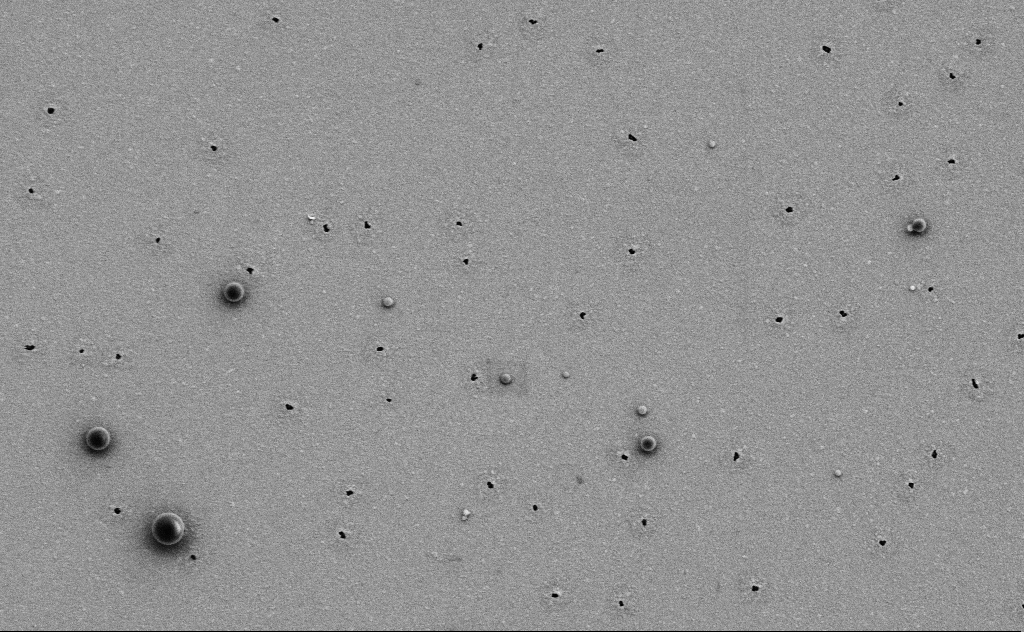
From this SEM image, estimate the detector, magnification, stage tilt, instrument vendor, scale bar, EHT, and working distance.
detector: SE2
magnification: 13.79 K X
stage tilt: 0°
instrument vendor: Zeiss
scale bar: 1000 nm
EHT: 3 kV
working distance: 10 mm